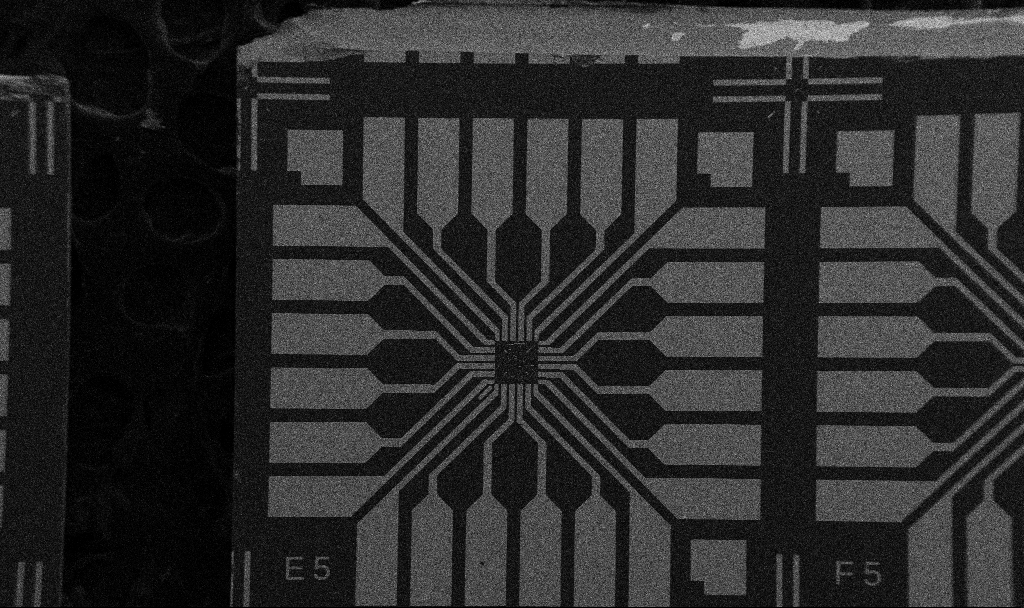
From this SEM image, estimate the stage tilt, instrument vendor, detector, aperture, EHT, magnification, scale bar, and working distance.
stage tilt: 0°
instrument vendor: Zeiss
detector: SE2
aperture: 30 µm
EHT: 5 kV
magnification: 0.1 K X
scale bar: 200000 nm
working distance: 10.7 mm